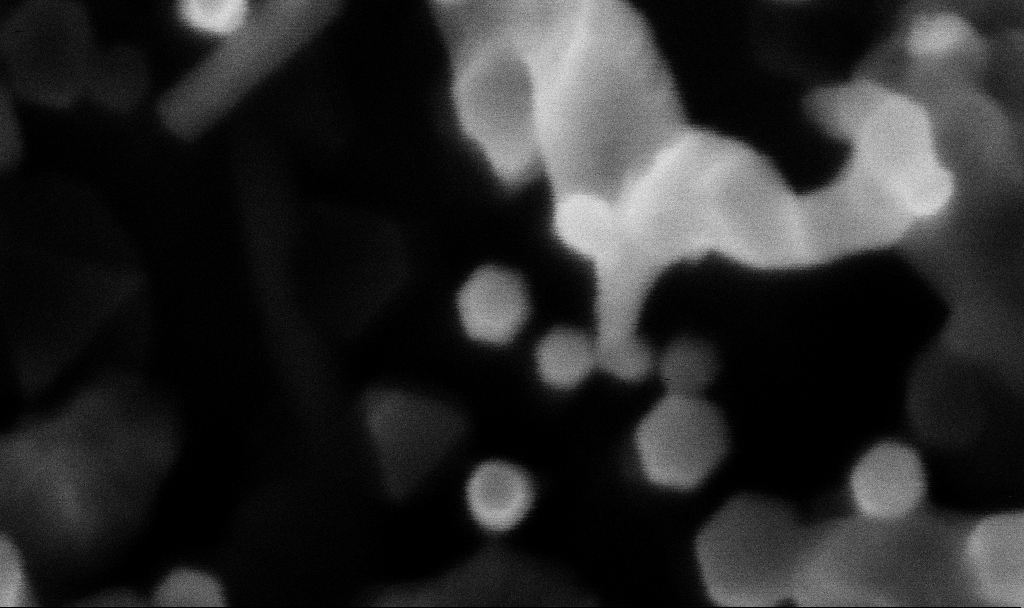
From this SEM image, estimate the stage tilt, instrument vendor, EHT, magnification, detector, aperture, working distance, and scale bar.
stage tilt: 0°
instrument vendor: Zeiss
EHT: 3 kV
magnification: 700.58 K X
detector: InLens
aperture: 30 µm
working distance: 3.1 mm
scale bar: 100 nm